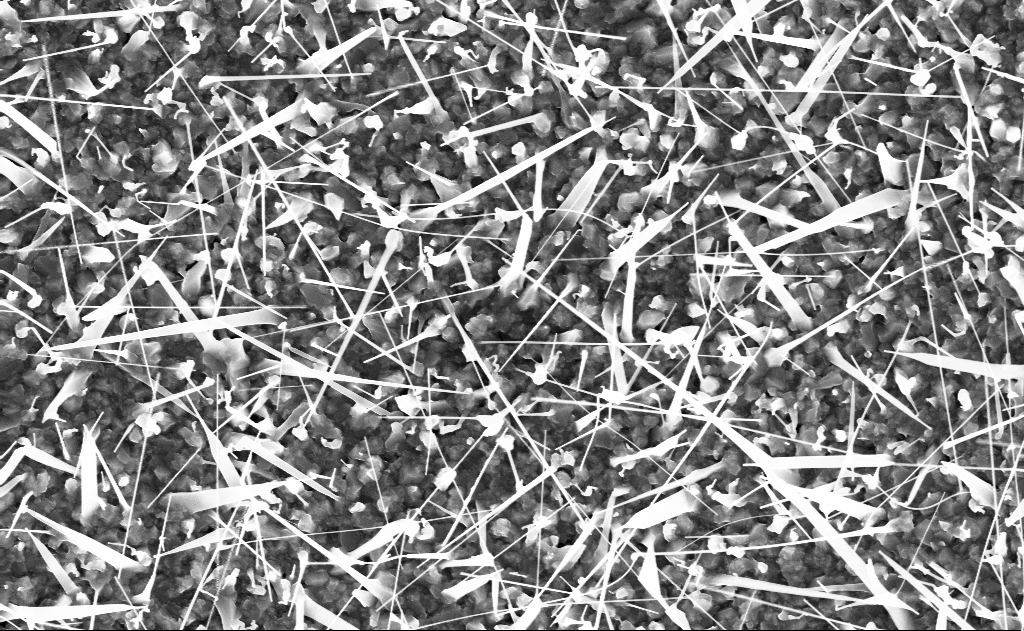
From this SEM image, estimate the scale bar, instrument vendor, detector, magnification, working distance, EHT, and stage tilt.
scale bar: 2000 nm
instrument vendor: Zeiss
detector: InLens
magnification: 20 K X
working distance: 9 mm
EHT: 10 kV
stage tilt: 0°